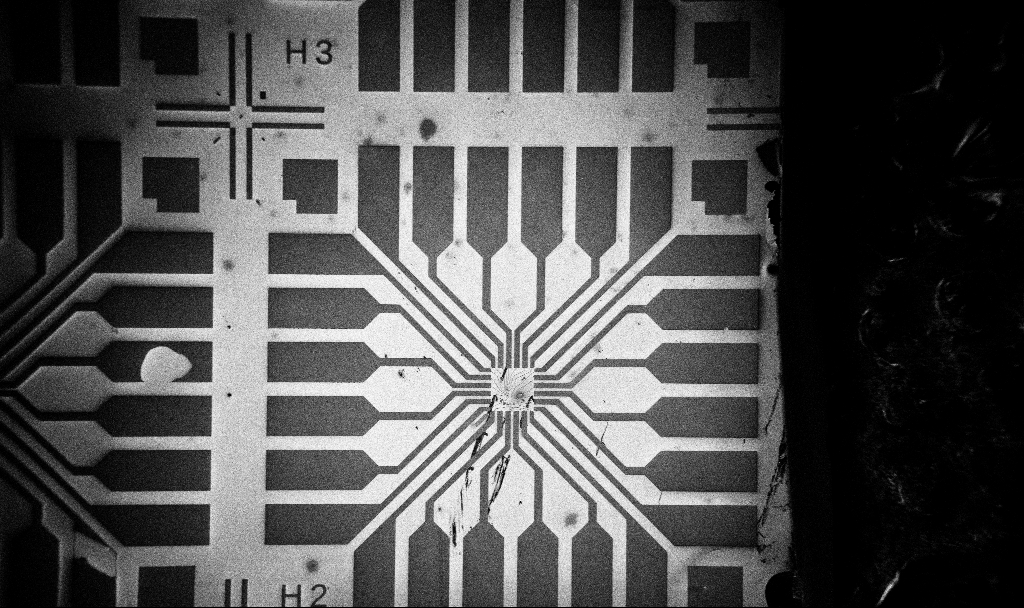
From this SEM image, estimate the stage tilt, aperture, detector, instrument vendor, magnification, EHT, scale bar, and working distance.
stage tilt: -0°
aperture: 30 µm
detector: InLens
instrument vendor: Zeiss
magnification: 0.1 K X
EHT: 5 kV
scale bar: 200000 nm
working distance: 10.7 mm